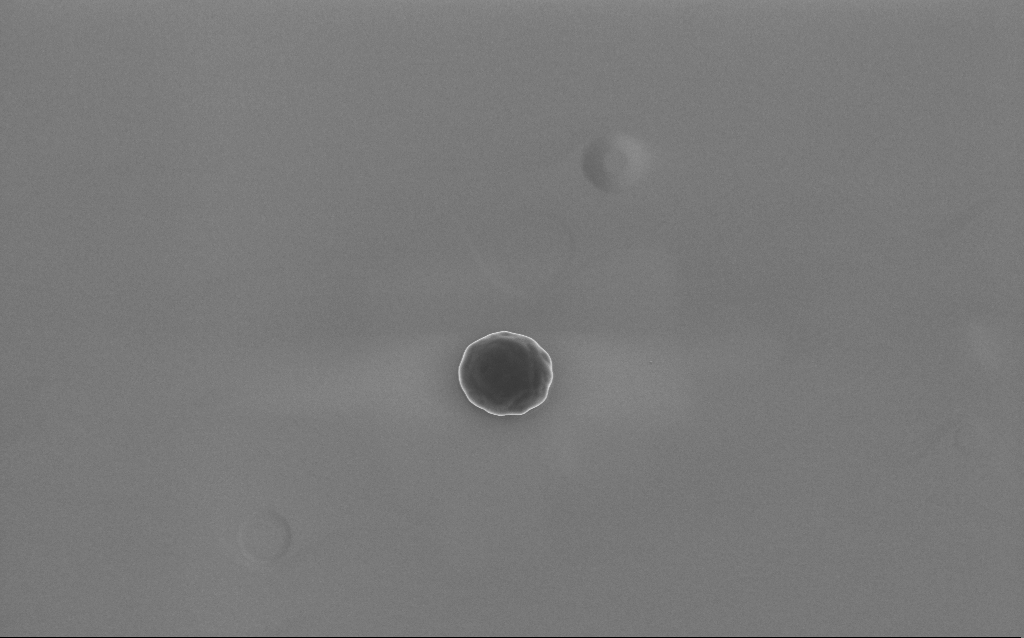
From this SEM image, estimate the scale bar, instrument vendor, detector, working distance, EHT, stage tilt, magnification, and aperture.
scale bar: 2000 nm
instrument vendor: Zeiss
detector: InLens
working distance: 4 mm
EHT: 5 kV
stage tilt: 20°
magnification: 13.37 K X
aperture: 30 µm